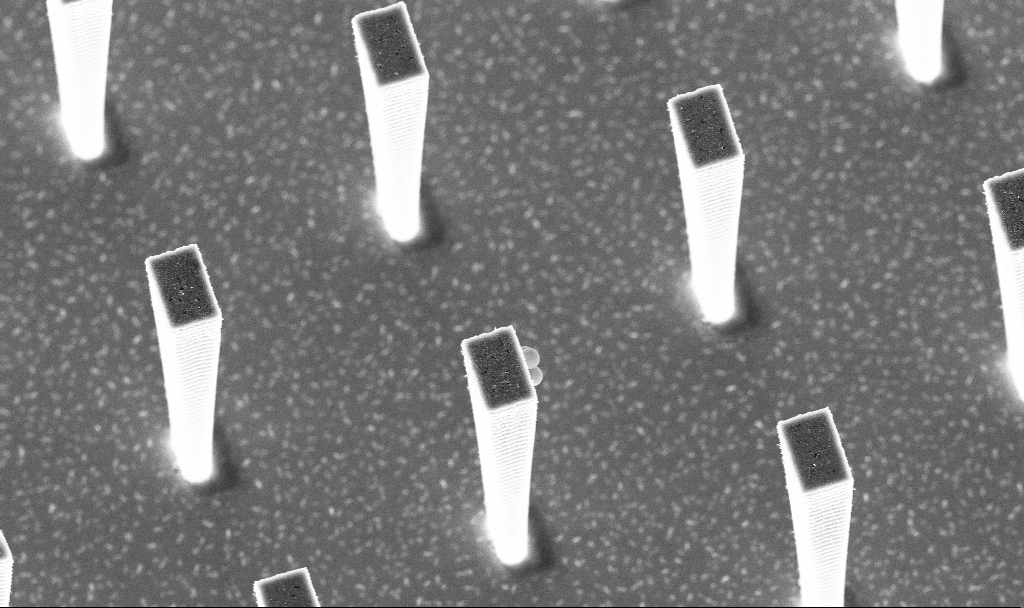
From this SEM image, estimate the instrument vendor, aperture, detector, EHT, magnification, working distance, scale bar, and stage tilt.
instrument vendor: Zeiss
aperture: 30 µm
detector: InLens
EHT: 5 kV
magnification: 10.88 K X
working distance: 5.2 mm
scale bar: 2000 nm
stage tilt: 20°